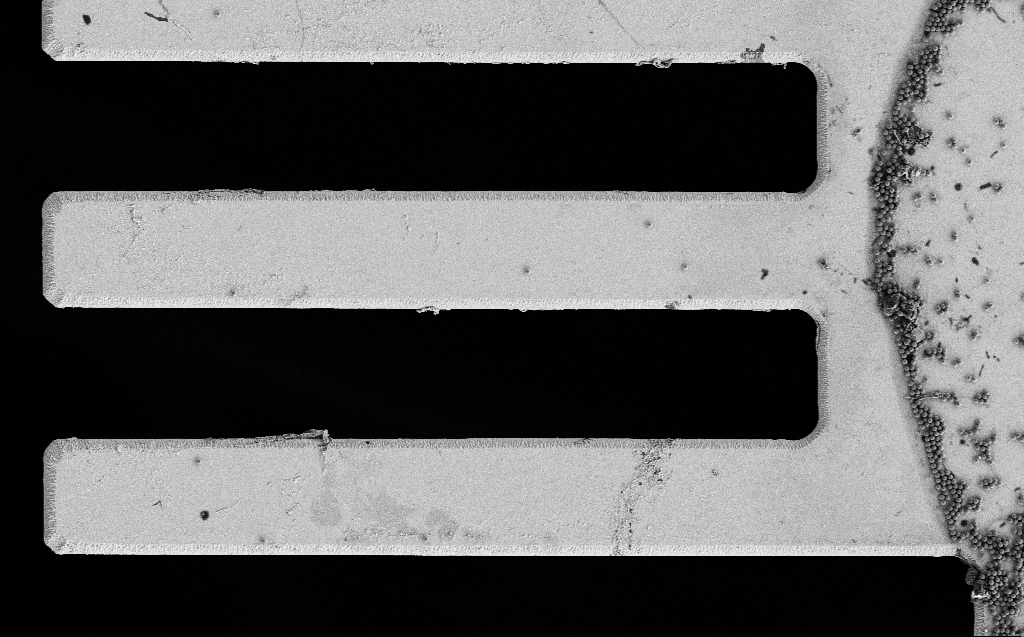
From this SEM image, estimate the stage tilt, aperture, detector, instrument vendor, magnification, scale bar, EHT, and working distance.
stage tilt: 0°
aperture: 30 µm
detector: SE2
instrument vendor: Zeiss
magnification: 2.28 K X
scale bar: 20000 nm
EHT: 3 kV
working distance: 7 mm